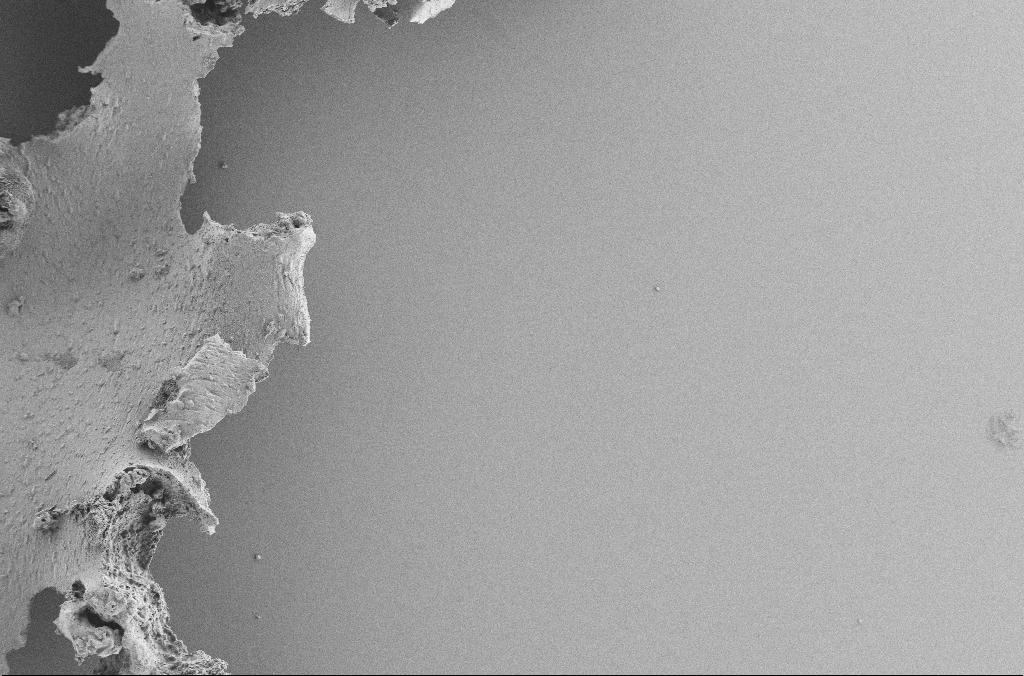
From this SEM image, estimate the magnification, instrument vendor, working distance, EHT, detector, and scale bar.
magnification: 0.25 K X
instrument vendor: Zeiss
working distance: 3.6 mm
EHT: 2 kV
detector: SE2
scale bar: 100000 nm